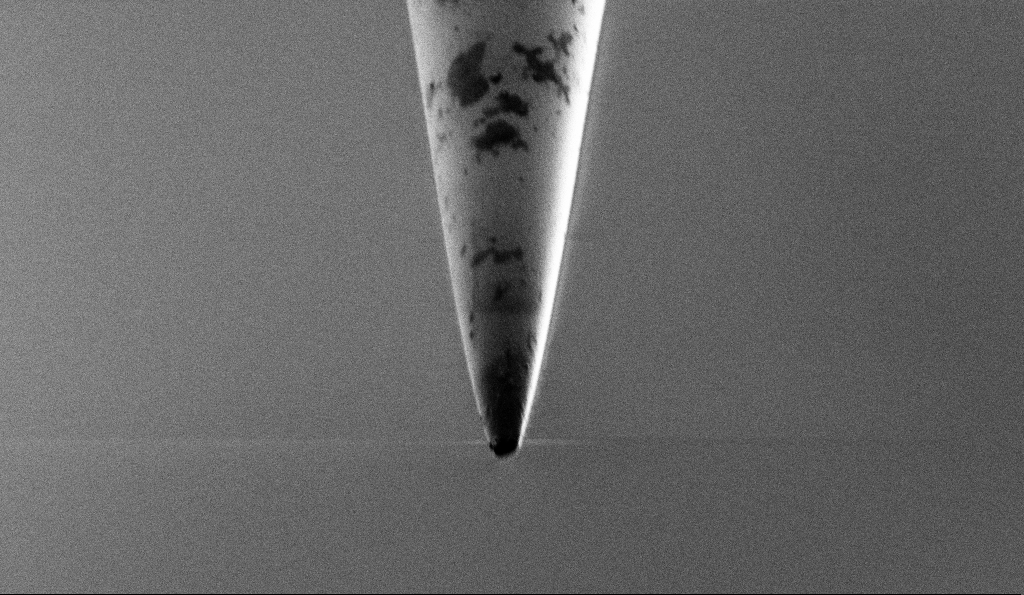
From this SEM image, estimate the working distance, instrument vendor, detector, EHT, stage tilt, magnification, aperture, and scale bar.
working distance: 7.8 mm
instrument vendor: Zeiss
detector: SE2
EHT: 1 kV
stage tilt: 45°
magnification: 5 K X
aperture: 30 µm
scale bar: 10000 nm